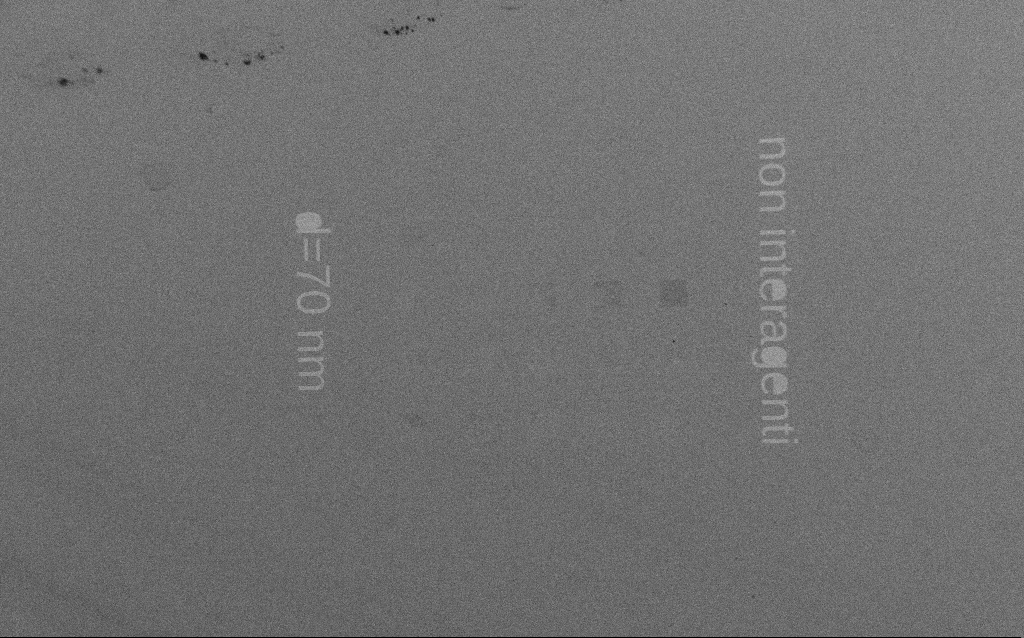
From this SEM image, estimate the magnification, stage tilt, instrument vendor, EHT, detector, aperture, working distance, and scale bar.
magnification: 0.237 K X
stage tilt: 0°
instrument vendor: Zeiss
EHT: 1.5 kV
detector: SE2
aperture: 30 µm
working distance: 5.8 mm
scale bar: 200000 nm